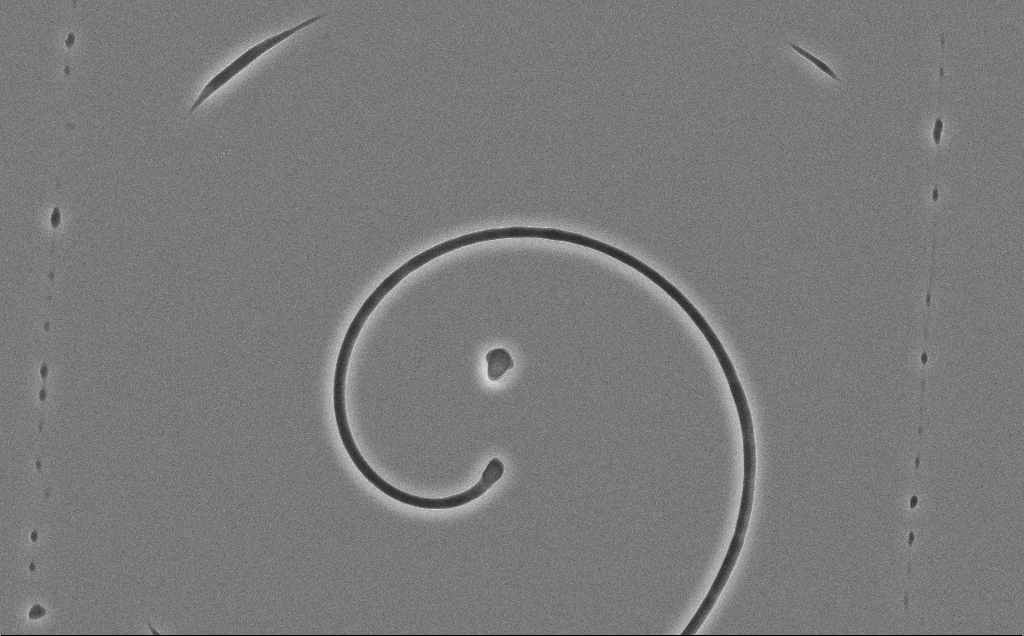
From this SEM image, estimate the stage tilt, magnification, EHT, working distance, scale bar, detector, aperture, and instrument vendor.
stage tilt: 0°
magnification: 3.81 K X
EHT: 10 kV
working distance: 12 mm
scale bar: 10000 nm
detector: SE2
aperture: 30 µm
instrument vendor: Zeiss